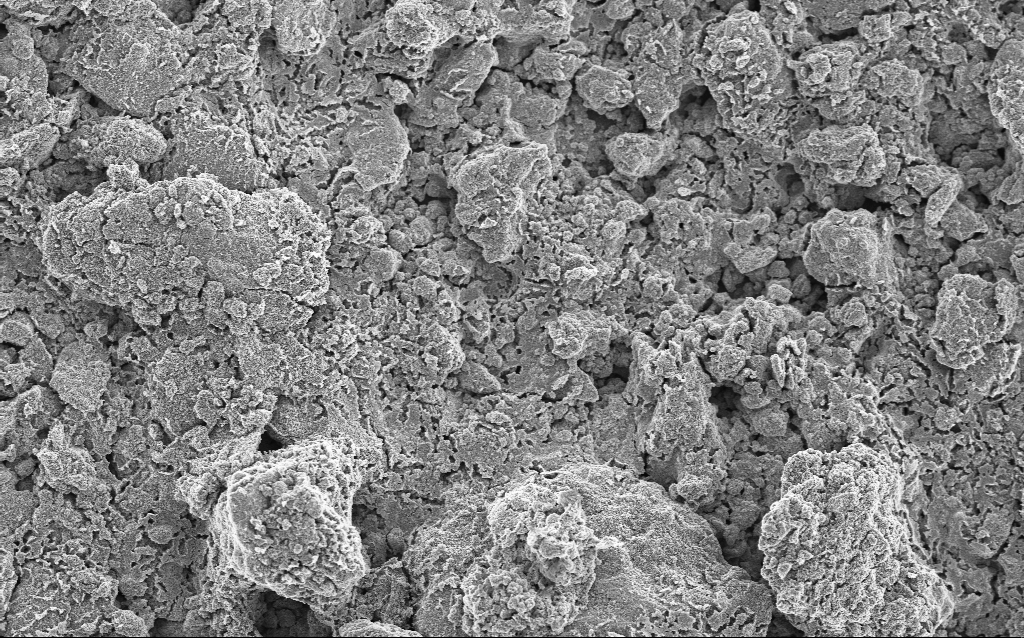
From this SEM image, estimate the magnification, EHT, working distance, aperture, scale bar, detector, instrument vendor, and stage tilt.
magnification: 1.23 K X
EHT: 5 kV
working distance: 4.4 mm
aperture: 30 µm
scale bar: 20000 nm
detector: InLens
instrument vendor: Zeiss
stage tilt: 0°